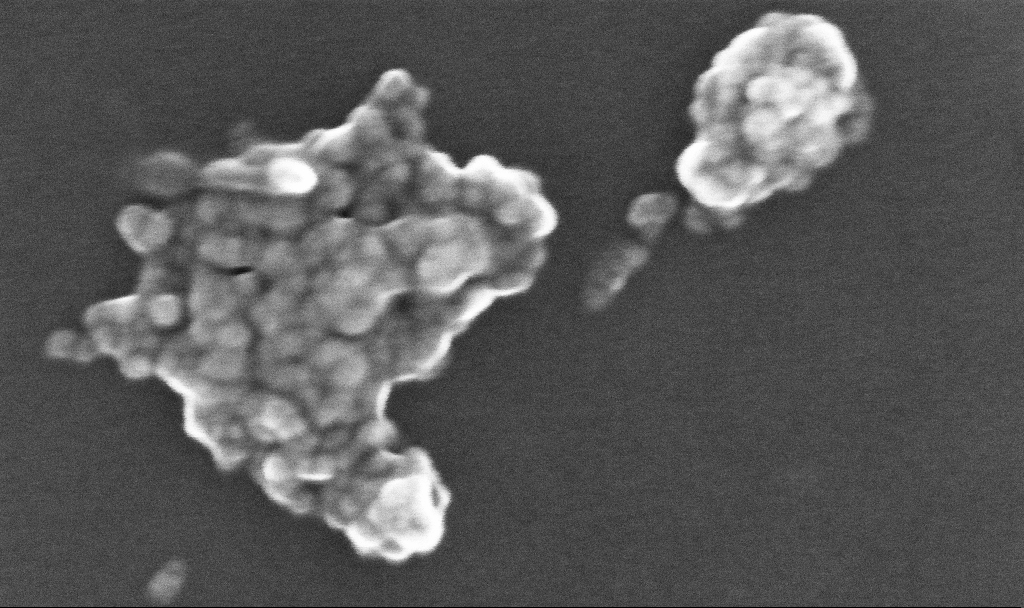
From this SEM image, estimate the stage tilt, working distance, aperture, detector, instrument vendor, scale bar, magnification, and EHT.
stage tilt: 0°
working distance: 5.2 mm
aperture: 30 µm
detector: InLens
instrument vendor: Zeiss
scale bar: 200 nm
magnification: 359.18 K X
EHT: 10 kV